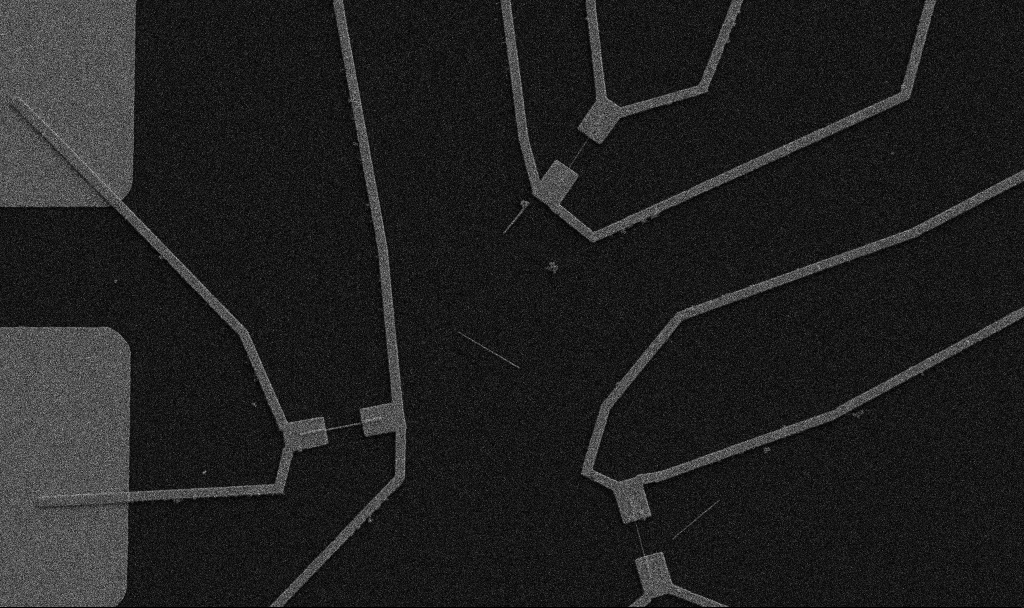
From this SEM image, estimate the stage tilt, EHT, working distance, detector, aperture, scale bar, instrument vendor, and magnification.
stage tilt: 0°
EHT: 5 kV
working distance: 10.7 mm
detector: SE2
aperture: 30 µm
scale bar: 10000 nm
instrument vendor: Zeiss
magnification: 5 K X